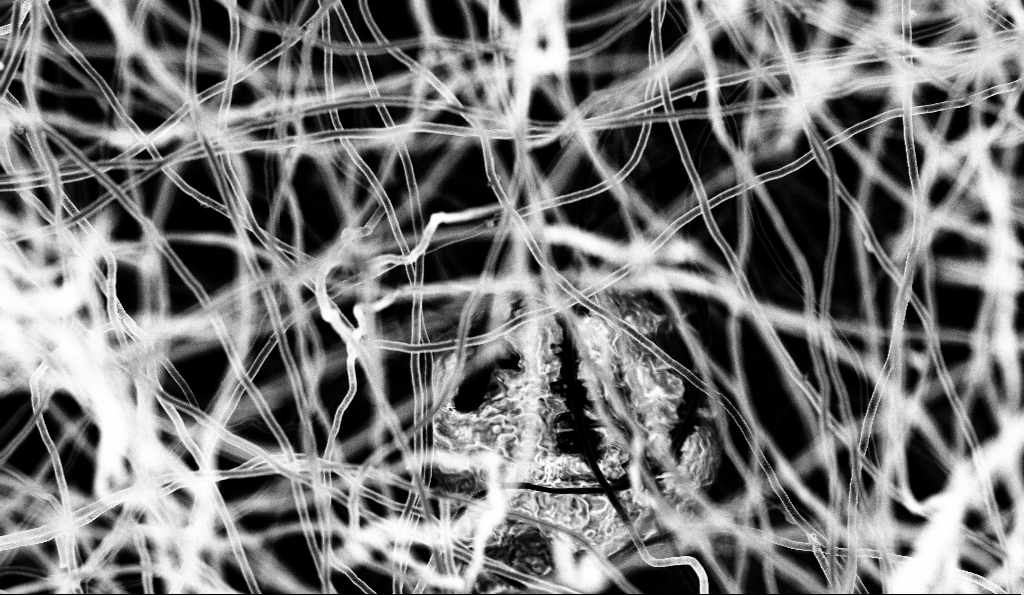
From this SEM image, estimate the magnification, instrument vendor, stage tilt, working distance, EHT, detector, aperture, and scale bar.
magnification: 5 K X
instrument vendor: Zeiss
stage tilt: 0°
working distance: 5 mm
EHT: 3 kV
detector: InLens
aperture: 30 µm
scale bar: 10000 nm